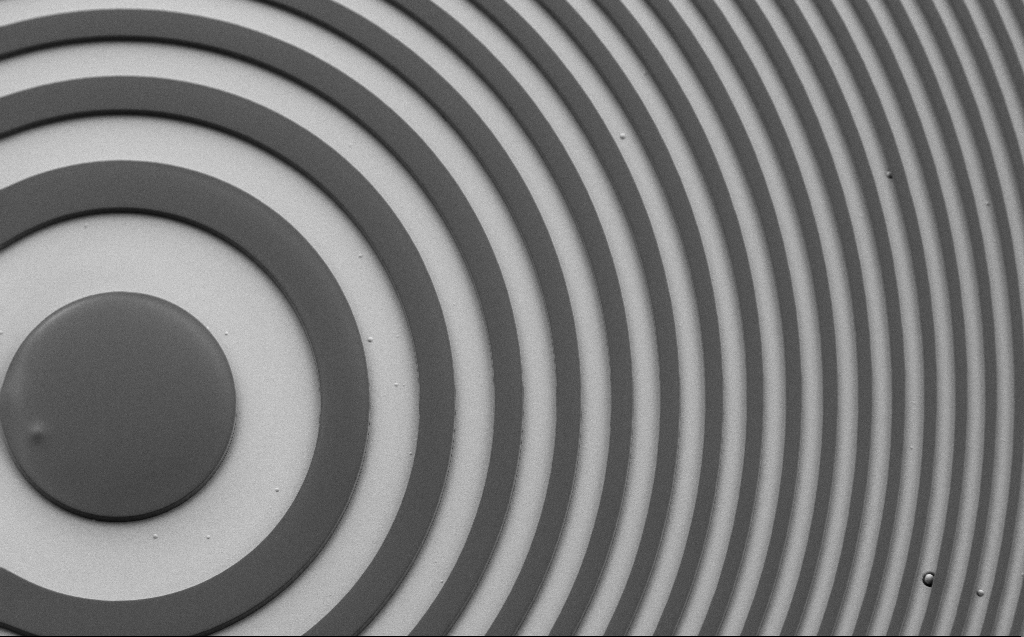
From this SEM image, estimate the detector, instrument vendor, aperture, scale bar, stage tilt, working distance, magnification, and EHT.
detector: SE2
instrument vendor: Zeiss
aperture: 30 µm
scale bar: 20000 nm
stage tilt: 30°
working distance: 6 mm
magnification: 1.3 K X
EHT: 1.1 kV